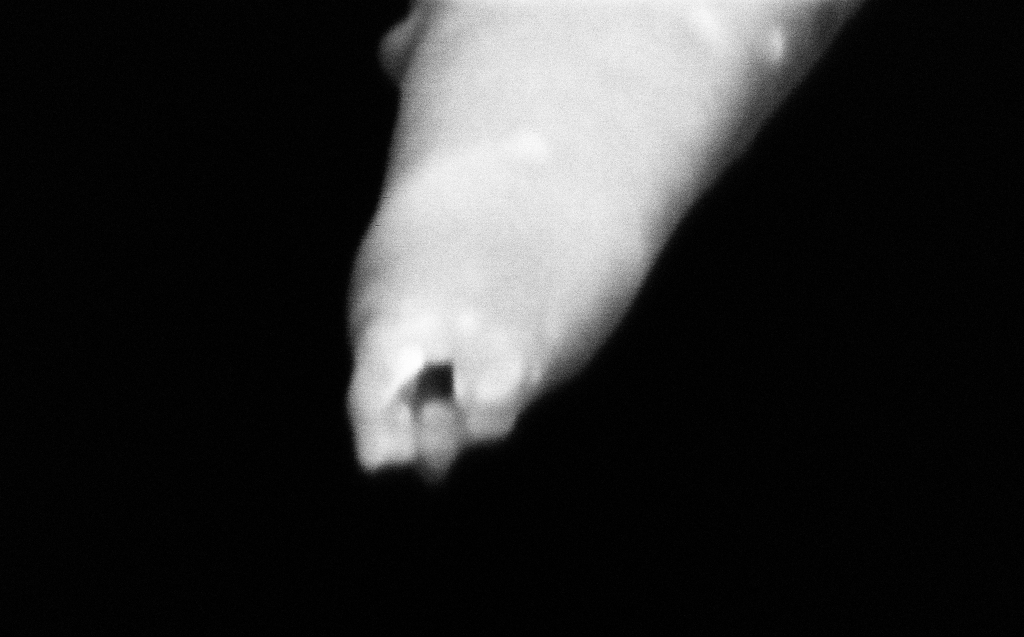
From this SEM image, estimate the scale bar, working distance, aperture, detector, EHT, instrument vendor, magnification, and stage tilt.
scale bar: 100 nm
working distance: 4 mm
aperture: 30 µm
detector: InLens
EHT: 2 kV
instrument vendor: Zeiss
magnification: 500 K X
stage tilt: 45°